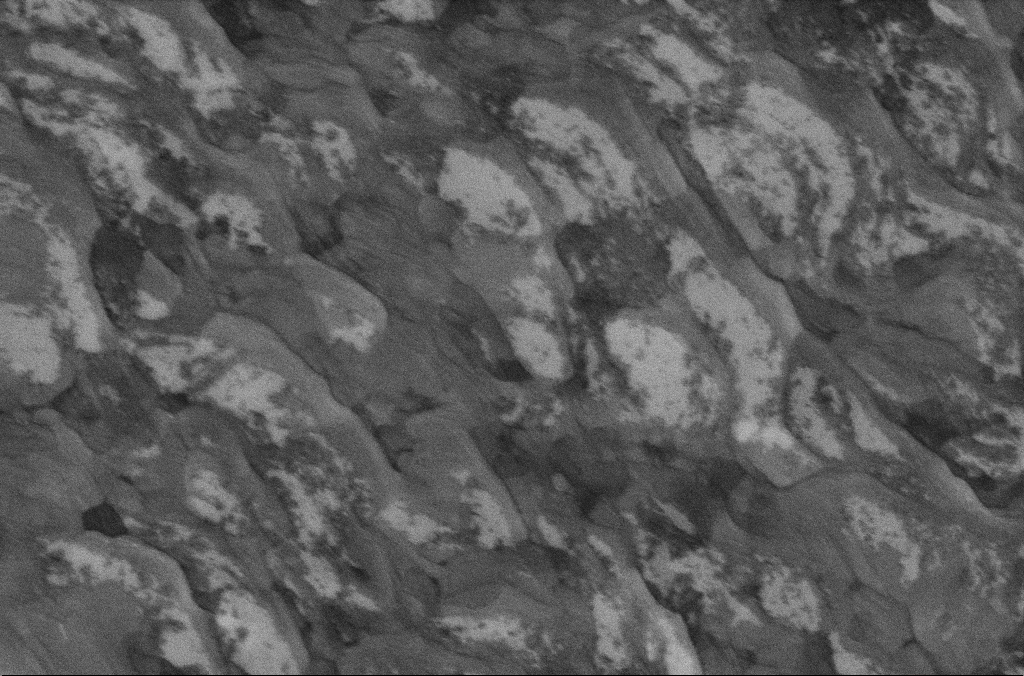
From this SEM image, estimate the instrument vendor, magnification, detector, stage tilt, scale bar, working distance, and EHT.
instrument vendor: Zeiss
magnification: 50 K X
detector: InLens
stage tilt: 0°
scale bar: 1000 nm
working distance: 6.7 mm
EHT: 5 kV